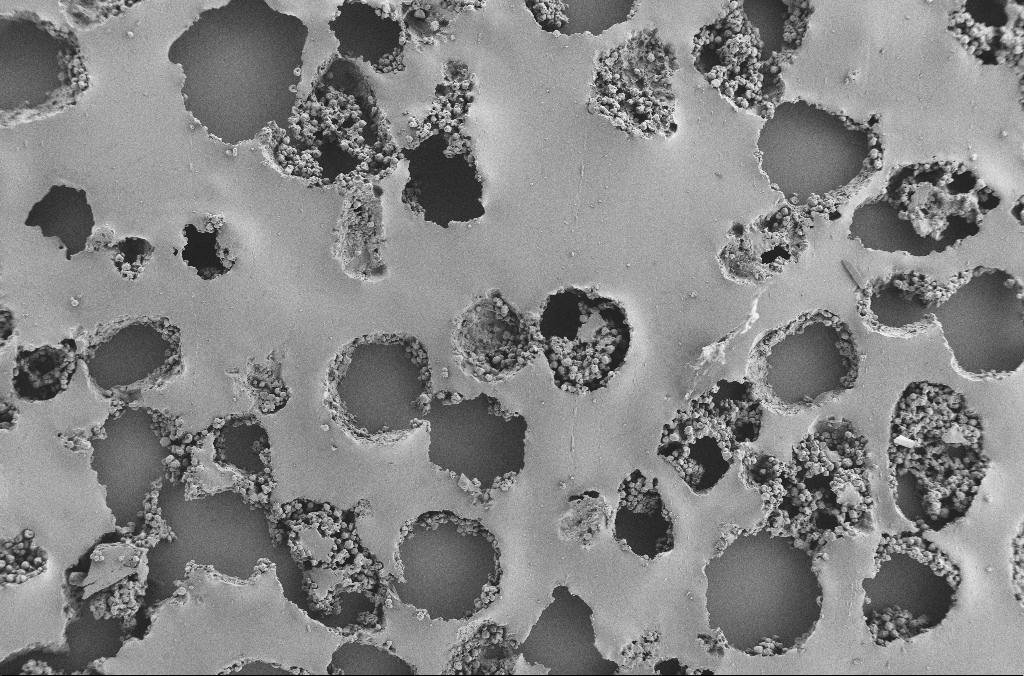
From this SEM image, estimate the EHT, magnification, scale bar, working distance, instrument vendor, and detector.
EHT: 2 kV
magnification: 0.2 K X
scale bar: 200000 nm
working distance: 3.7 mm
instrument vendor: Zeiss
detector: SE2